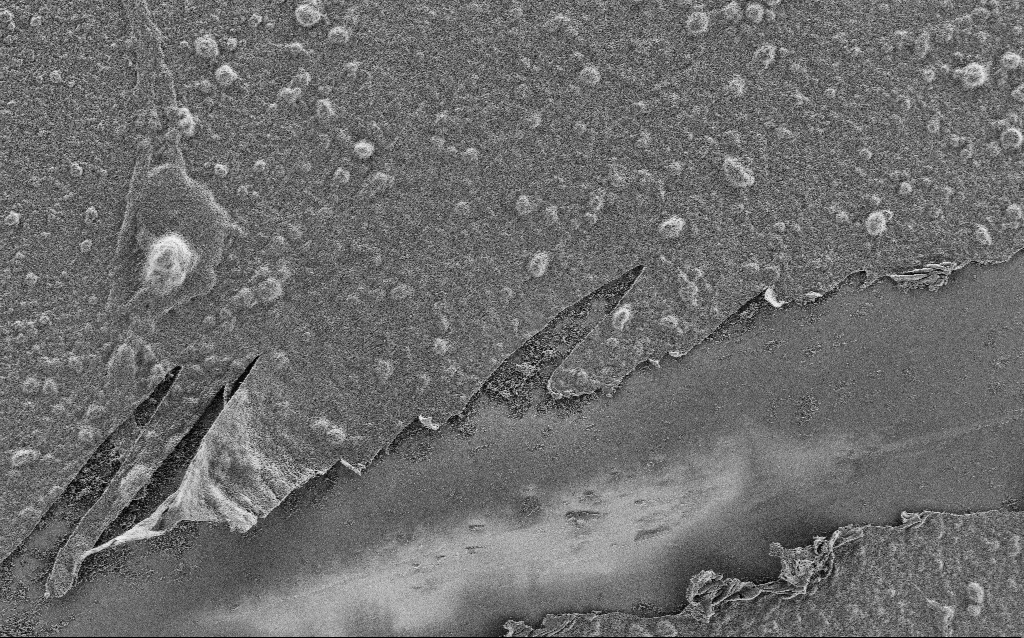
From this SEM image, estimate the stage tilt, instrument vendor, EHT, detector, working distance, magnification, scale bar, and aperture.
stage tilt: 0°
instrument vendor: Zeiss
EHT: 1 kV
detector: SE2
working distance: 4 mm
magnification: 2.5 K X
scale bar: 10000 nm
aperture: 30 µm